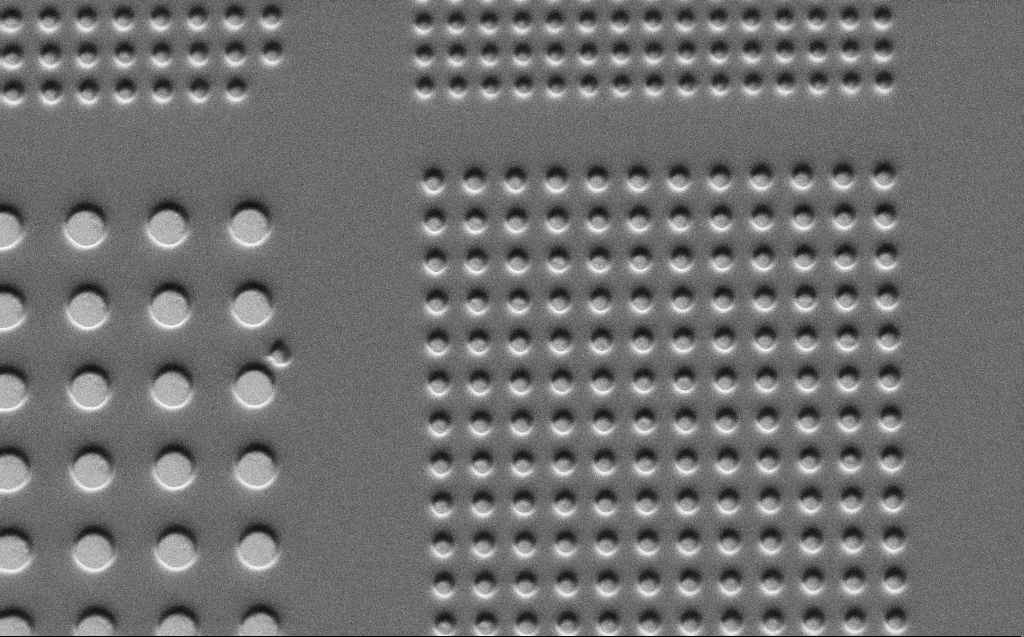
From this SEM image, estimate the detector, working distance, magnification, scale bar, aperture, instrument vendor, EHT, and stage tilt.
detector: SE2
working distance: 6 mm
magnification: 7.6 K X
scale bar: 2000 nm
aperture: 30 µm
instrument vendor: Zeiss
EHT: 3 kV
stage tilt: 45°